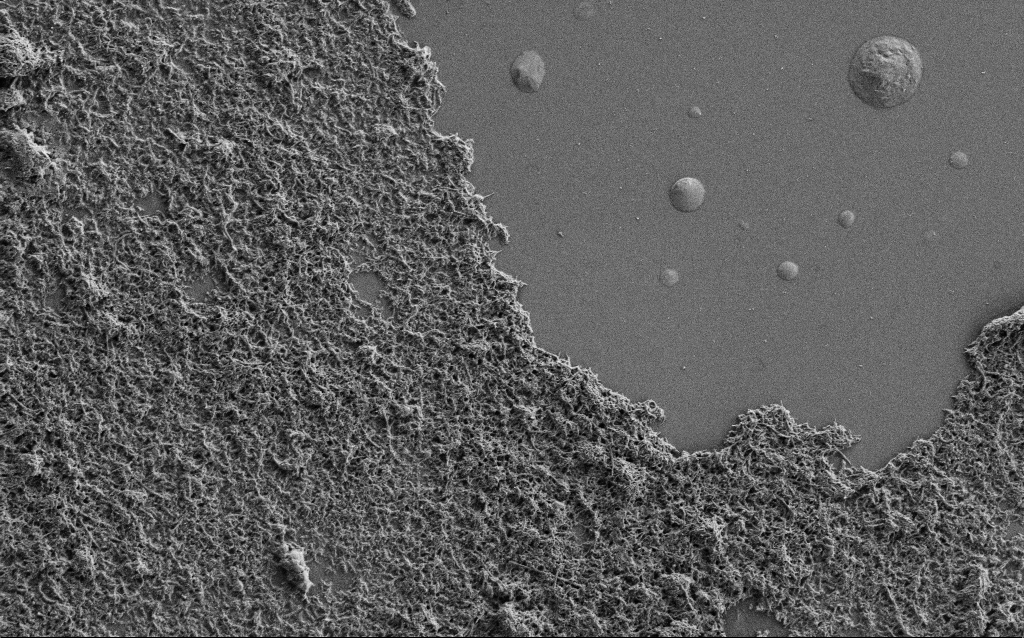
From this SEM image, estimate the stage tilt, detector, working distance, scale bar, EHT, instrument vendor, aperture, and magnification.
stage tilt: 0°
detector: SE2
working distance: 6 mm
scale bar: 2000 nm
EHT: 1 kV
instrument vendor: Zeiss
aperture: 30 µm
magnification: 10 K X